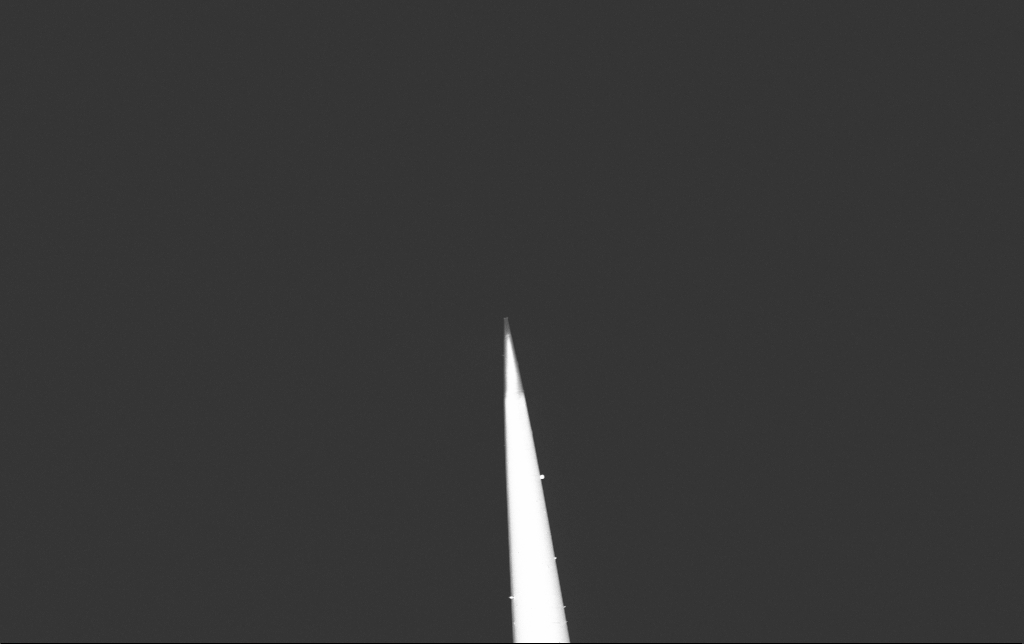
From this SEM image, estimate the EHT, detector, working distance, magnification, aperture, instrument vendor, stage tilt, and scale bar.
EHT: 2 kV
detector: InLens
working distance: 6.6 mm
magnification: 5 K X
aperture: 30 µm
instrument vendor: Zeiss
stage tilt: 0°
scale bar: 10000 nm